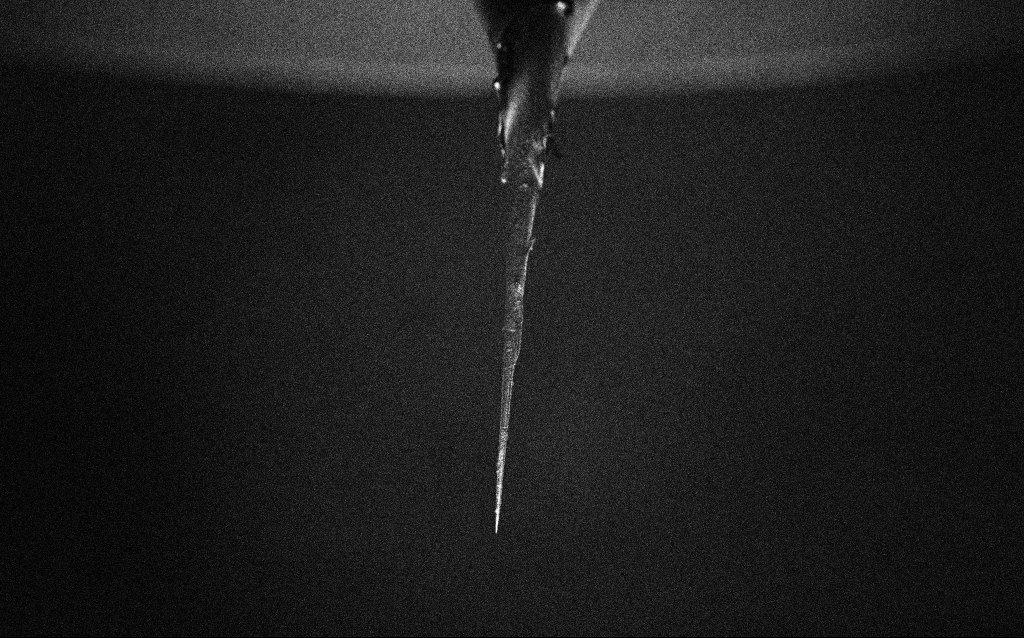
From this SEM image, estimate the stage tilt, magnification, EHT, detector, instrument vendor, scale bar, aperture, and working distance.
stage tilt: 43.9°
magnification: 0.1 K X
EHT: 5 kV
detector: InLens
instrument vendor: Zeiss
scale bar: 200000 nm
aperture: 30 µm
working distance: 8.3 mm